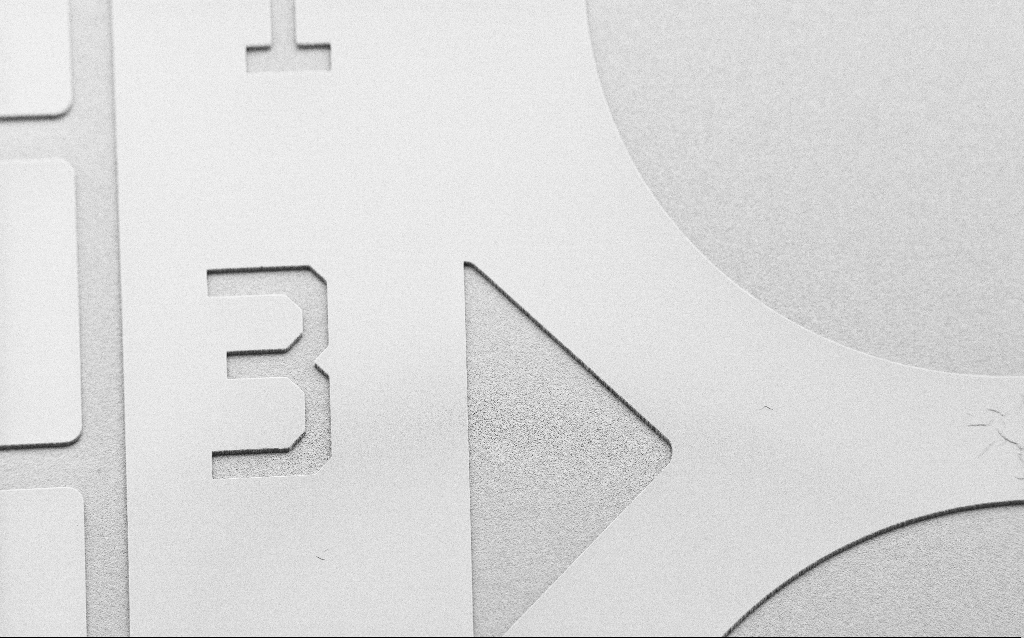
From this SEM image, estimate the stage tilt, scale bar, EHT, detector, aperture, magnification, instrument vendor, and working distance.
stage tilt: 45°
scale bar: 100000 nm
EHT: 2 kV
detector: SE2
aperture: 30 µm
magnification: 0.313 K X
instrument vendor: Zeiss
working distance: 8 mm